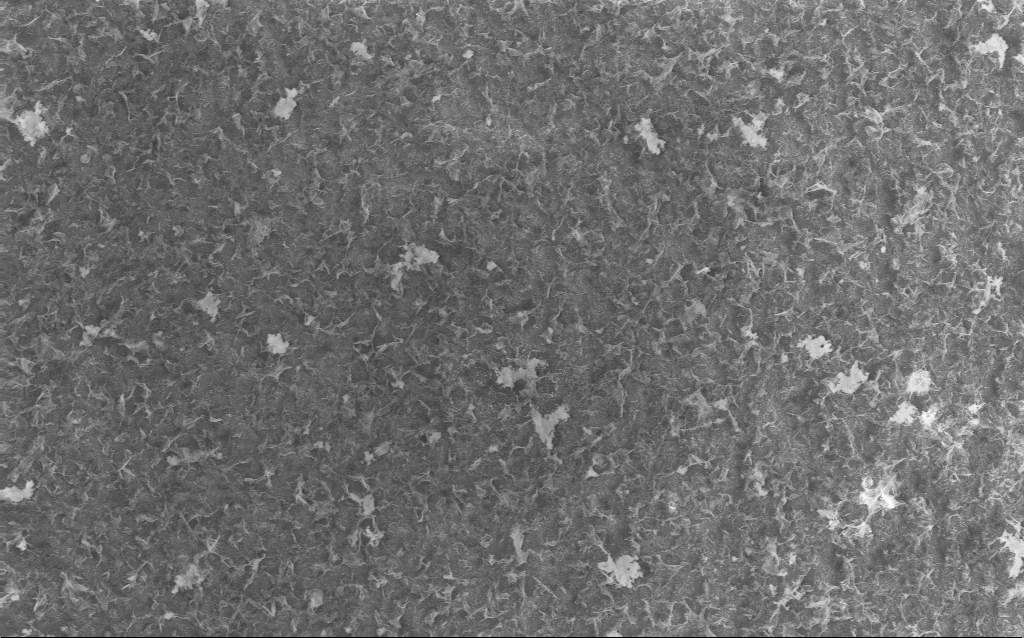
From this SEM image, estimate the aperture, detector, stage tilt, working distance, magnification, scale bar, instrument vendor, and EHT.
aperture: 30 µm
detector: InLens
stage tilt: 0°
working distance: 2.5 mm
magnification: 5 K X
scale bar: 10000 nm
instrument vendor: Zeiss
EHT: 10 kV